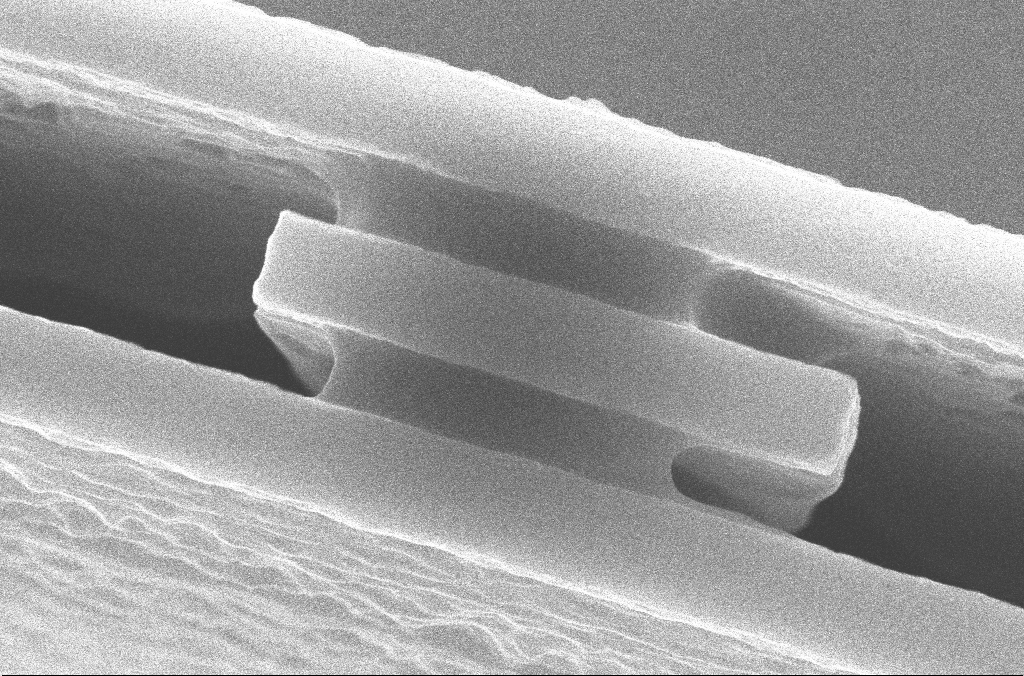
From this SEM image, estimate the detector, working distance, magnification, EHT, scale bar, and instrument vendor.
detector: InLens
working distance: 7.1 mm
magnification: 73.83 K X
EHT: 10 kV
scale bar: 200 nm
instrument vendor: Zeiss